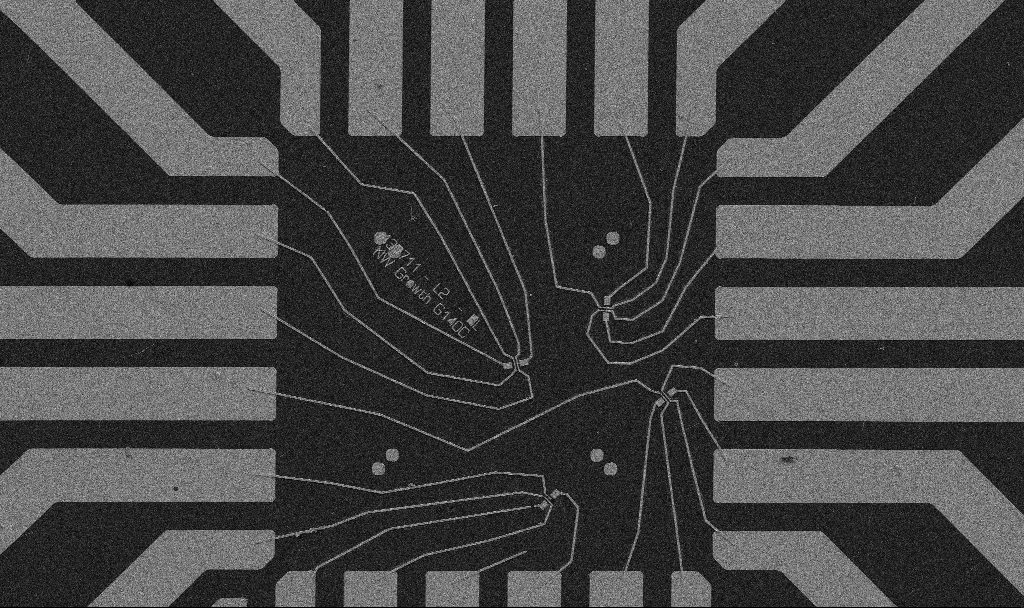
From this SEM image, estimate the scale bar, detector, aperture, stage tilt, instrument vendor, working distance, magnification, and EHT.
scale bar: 20000 nm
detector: SE2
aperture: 30 µm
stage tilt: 0°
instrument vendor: Zeiss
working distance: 10.7 mm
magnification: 1 K X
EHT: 5 kV